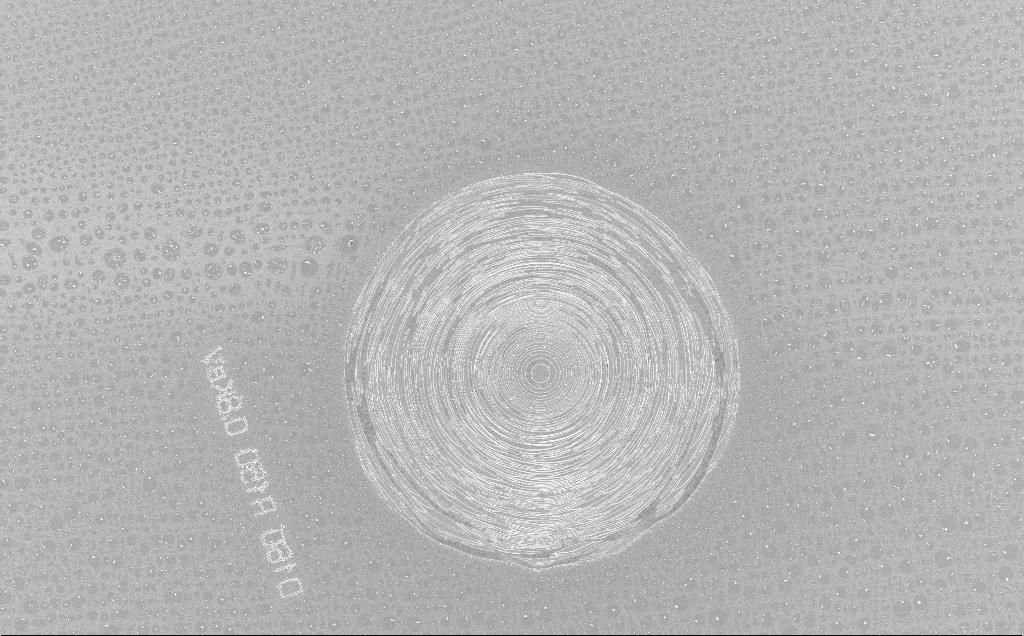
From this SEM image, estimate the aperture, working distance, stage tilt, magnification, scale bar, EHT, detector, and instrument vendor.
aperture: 30 µm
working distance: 6 mm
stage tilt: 0°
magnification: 0.927 K X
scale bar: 20000 nm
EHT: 5 kV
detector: InLens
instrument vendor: Zeiss